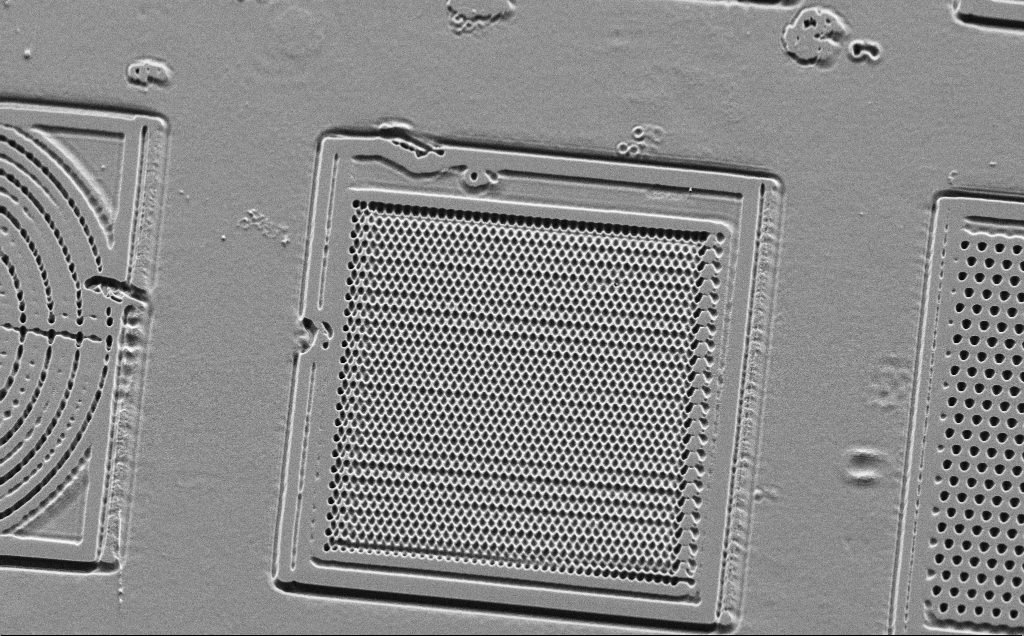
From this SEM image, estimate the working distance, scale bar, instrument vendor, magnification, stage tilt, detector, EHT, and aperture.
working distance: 10 mm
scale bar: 10000 nm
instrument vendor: Zeiss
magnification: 1.61 K X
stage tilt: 45°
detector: SE2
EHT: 5 kV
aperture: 30 µm